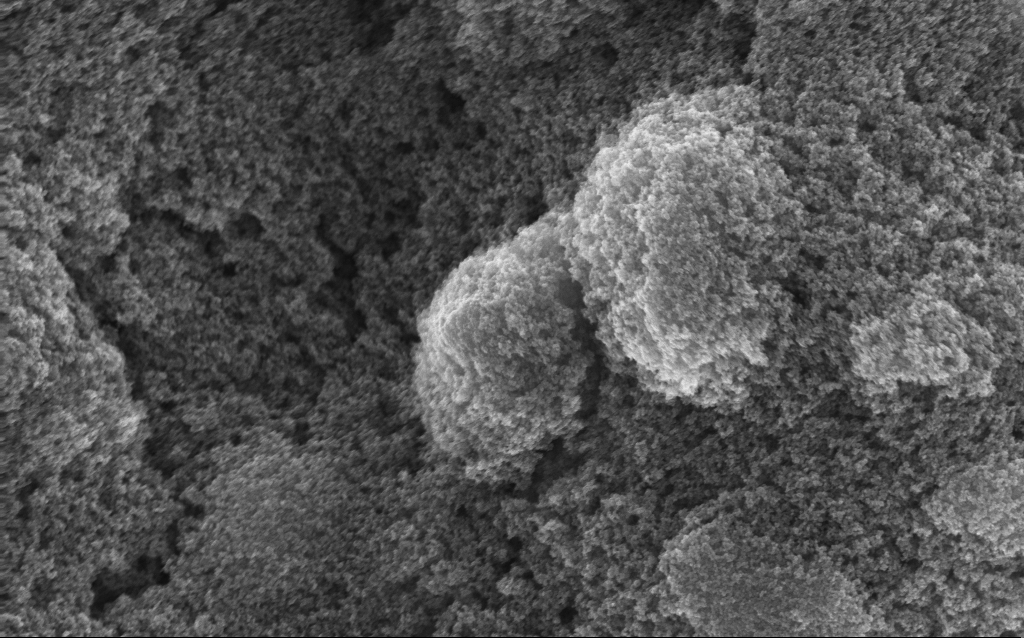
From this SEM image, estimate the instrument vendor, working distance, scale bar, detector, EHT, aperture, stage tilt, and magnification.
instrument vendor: Zeiss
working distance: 2.7 mm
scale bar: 1000 nm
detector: InLens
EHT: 10 kV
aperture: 20 µm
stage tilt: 0°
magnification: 37.88 K X